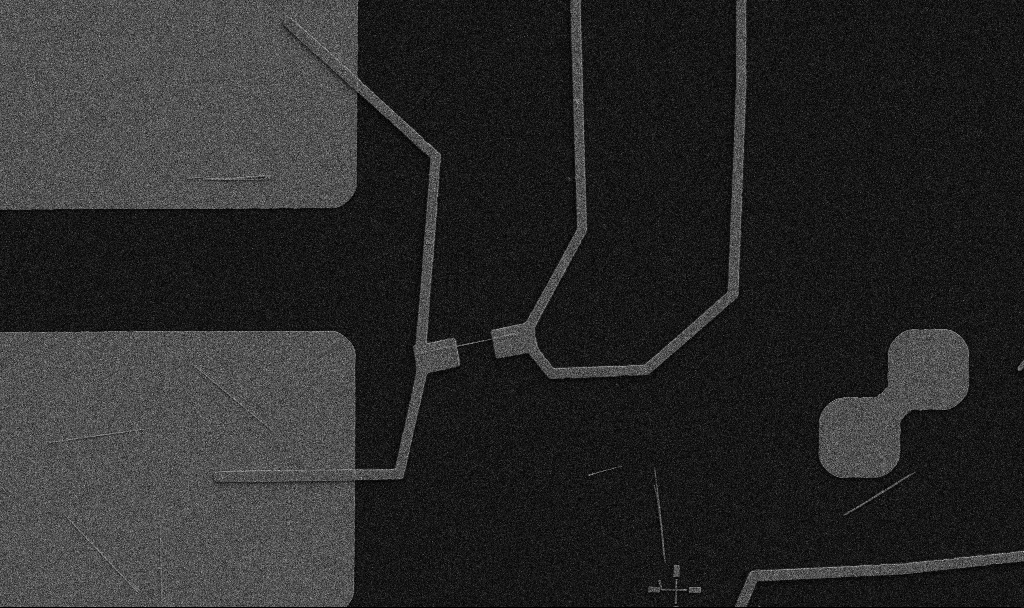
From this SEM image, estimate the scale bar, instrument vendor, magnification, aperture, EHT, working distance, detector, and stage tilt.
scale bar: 10000 nm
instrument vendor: Zeiss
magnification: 5 K X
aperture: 30 µm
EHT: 5 kV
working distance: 10.7 mm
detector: SE2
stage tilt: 0°